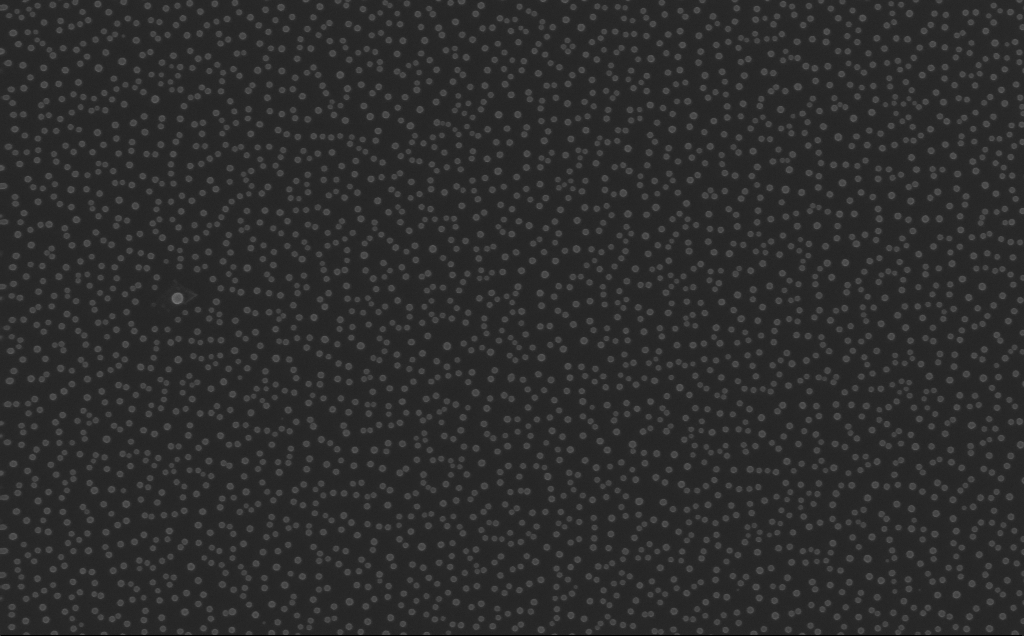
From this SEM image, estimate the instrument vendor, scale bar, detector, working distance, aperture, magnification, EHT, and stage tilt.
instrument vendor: Zeiss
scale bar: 200 nm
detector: InLens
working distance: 4 mm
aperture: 30 µm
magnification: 80 K X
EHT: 10 kV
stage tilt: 0°